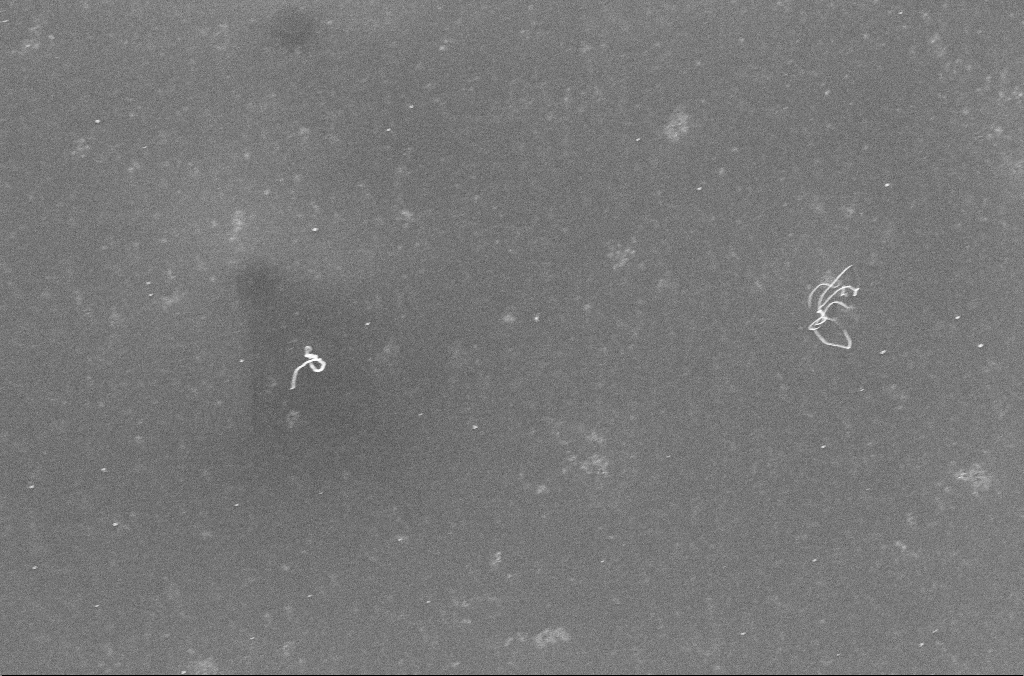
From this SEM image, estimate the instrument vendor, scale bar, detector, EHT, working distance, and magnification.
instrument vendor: Zeiss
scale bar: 1000 nm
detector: InLens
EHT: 10 kV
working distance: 3.2 mm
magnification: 36.58 K X